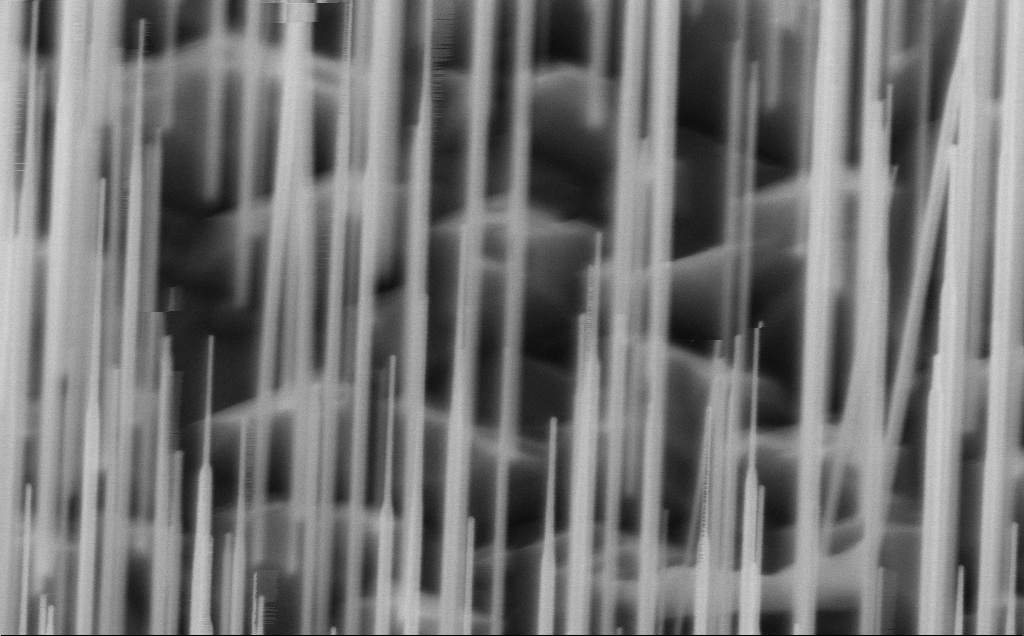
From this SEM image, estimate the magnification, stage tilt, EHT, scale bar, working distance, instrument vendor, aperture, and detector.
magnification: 80 K X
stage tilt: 45°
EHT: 10 kV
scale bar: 200 nm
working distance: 5 mm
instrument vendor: Zeiss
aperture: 30 µm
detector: InLens